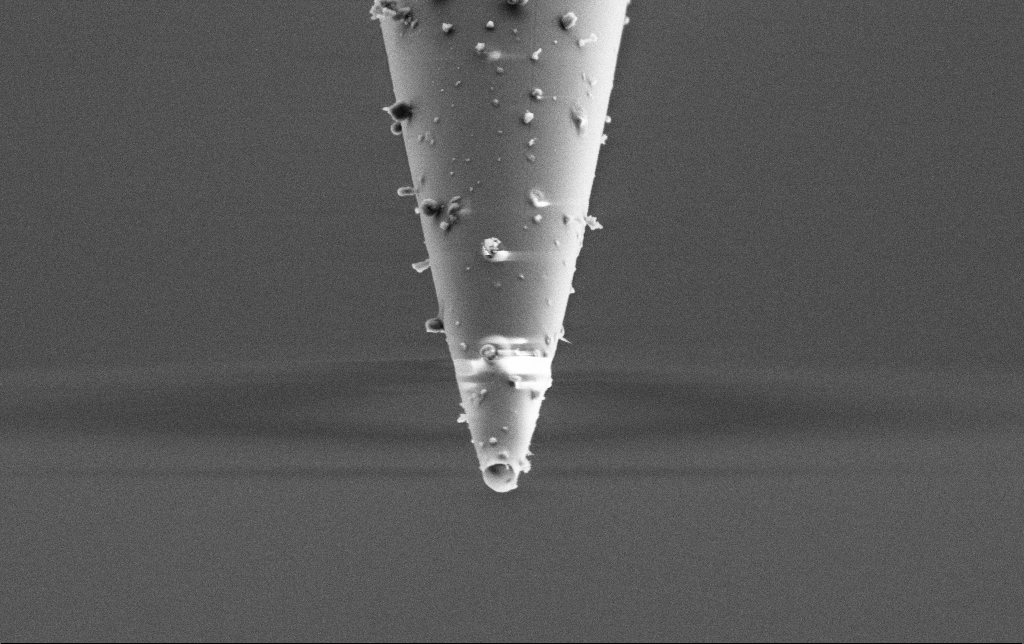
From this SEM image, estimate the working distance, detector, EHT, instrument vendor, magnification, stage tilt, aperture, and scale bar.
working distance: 7.4 mm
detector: SE2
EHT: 2 kV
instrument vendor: Zeiss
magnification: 25 K X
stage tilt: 45°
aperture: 30 µm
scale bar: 1000 nm